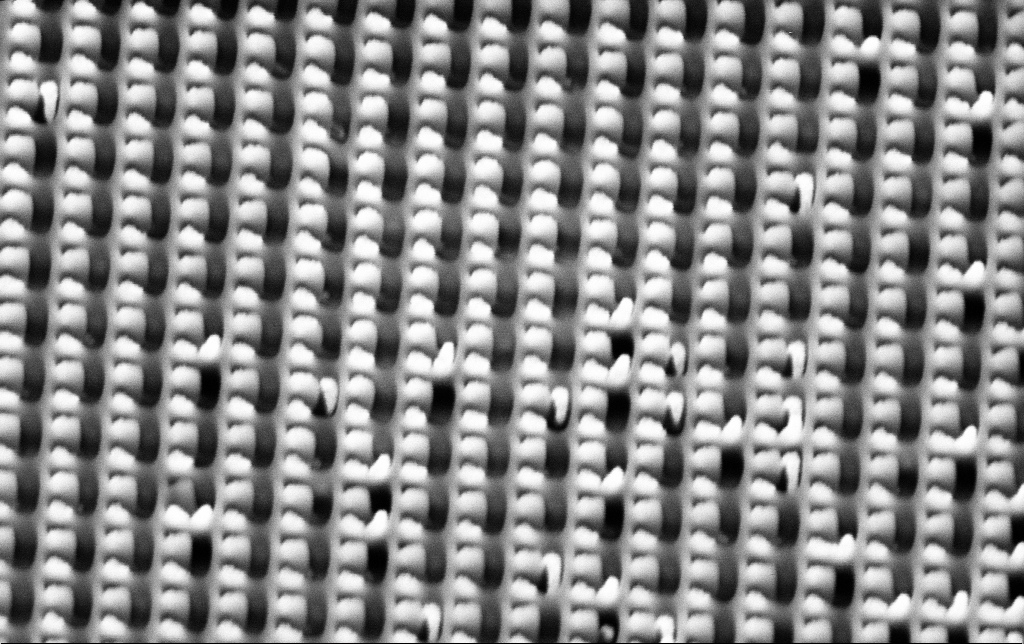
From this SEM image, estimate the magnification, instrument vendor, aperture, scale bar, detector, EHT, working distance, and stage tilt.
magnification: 46.28 K X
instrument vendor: Zeiss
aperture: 30 µm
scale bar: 1000 nm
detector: SE2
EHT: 3 kV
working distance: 10.4 mm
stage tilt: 45°